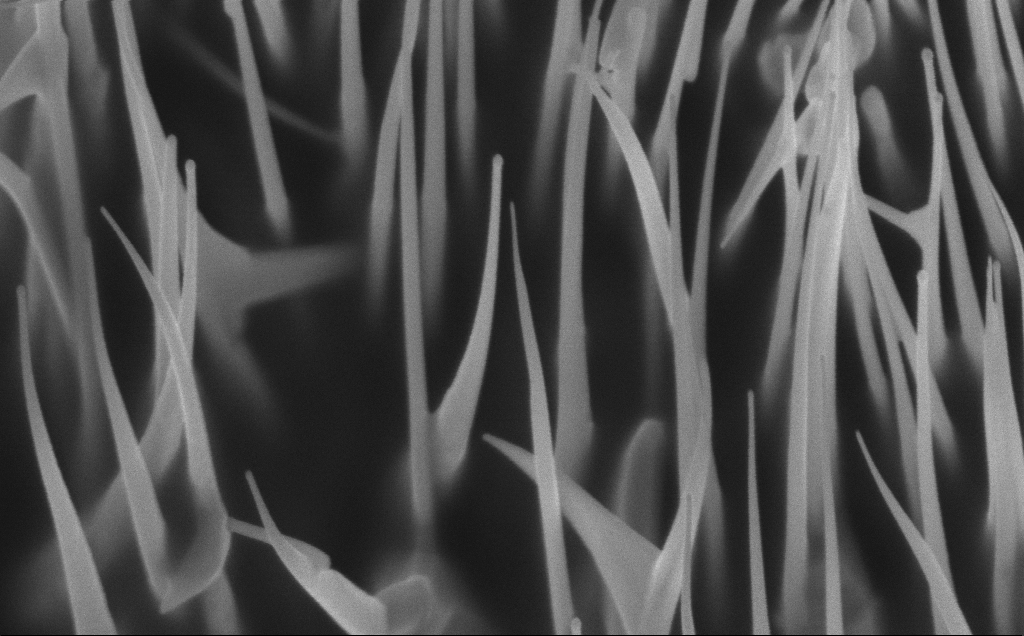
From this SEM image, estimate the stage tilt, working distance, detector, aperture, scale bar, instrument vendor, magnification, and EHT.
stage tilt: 30°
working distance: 7 mm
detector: InLens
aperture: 30 µm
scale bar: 1000 nm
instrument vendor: Zeiss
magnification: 71.19 K X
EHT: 5 kV